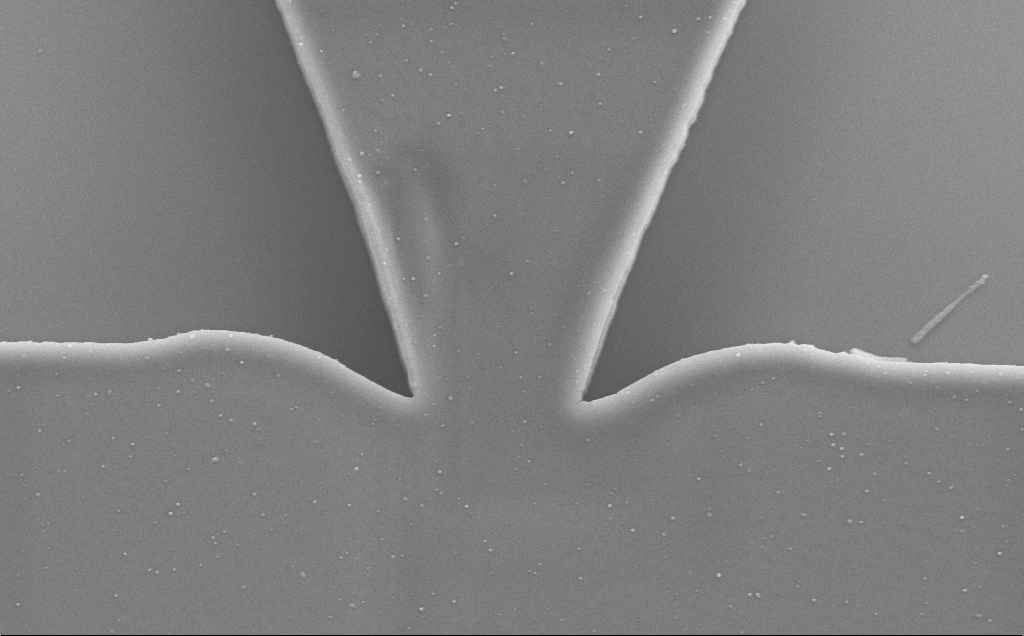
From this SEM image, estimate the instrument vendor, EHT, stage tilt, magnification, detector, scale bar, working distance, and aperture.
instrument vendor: Zeiss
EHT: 5 kV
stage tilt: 0°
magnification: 18.31 K X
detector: SE2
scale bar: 2000 nm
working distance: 9 mm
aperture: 30 µm